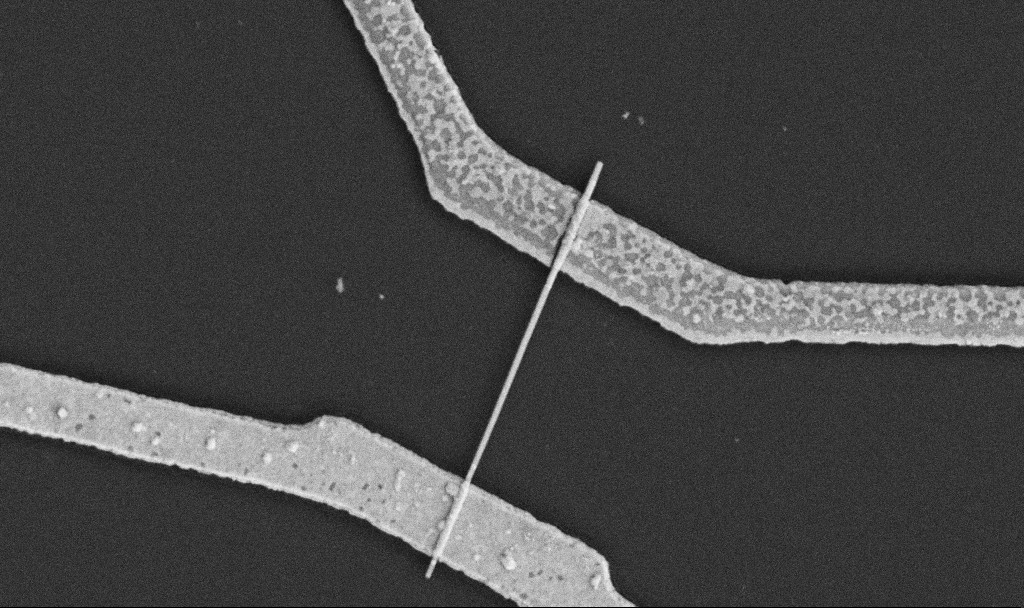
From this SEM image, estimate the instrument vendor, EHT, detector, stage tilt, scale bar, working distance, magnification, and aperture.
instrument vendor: Zeiss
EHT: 5 kV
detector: SE2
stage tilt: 0°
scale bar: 1000 nm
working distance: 10.6 mm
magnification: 30 K X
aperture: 30 µm